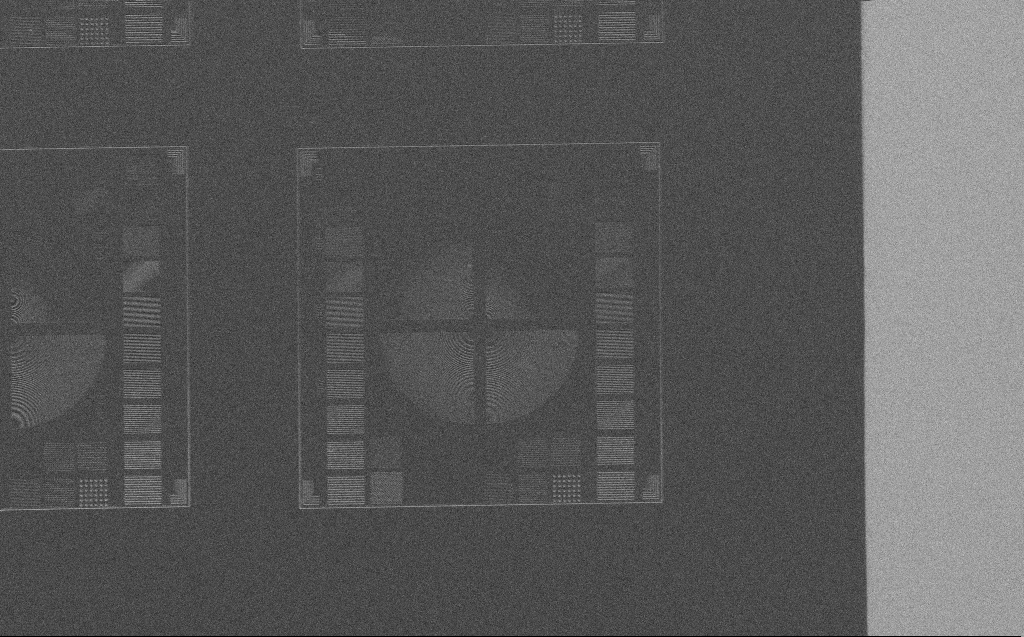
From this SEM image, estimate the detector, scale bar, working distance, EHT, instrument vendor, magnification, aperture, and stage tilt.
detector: SE2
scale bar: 100000 nm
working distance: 7 mm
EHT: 5 kV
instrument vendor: Zeiss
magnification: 0.452 K X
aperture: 30 µm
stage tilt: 0°